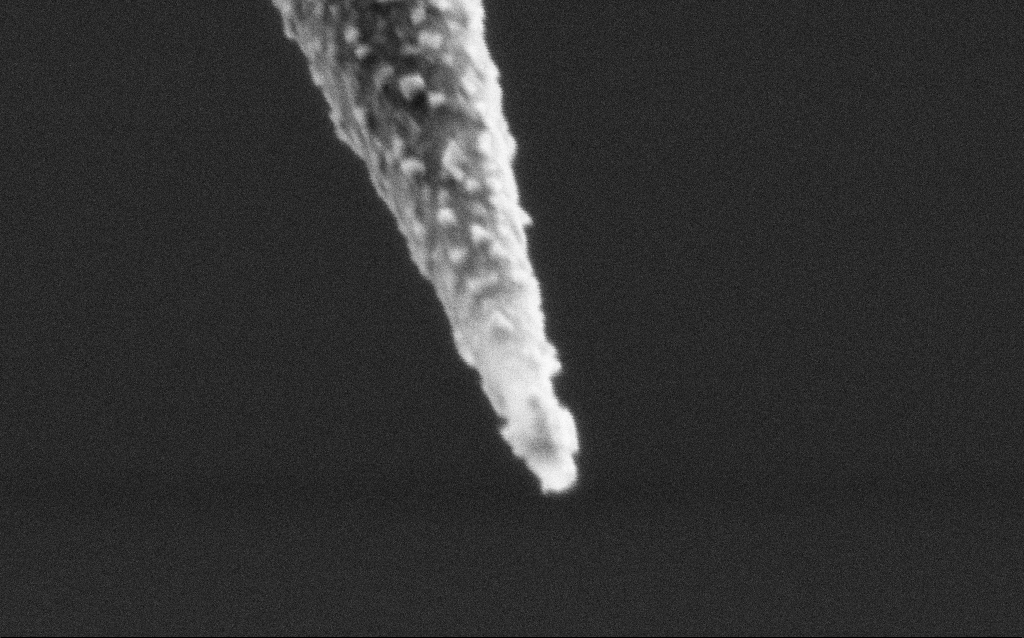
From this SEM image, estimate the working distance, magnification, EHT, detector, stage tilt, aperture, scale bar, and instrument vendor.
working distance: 7.8 mm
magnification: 150 K X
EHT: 2 kV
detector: SE2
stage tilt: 45°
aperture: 30 µm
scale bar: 100 nm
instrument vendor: Zeiss